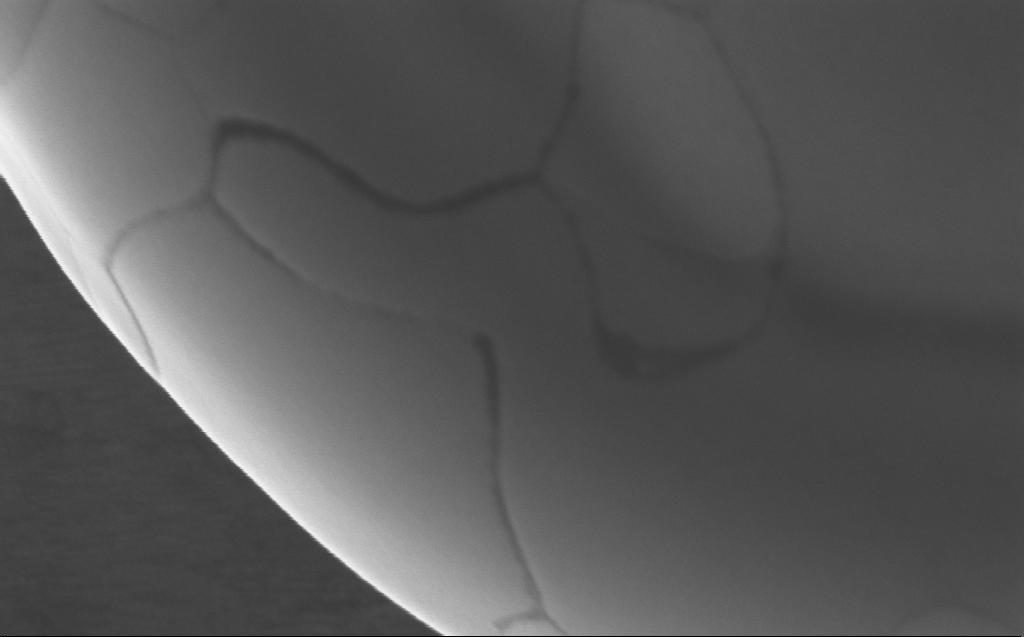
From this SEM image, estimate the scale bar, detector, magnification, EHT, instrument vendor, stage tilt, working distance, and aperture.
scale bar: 200 nm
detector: InLens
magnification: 227.22 K X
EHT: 5 kV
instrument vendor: Zeiss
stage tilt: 0°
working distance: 2 mm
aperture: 30 µm